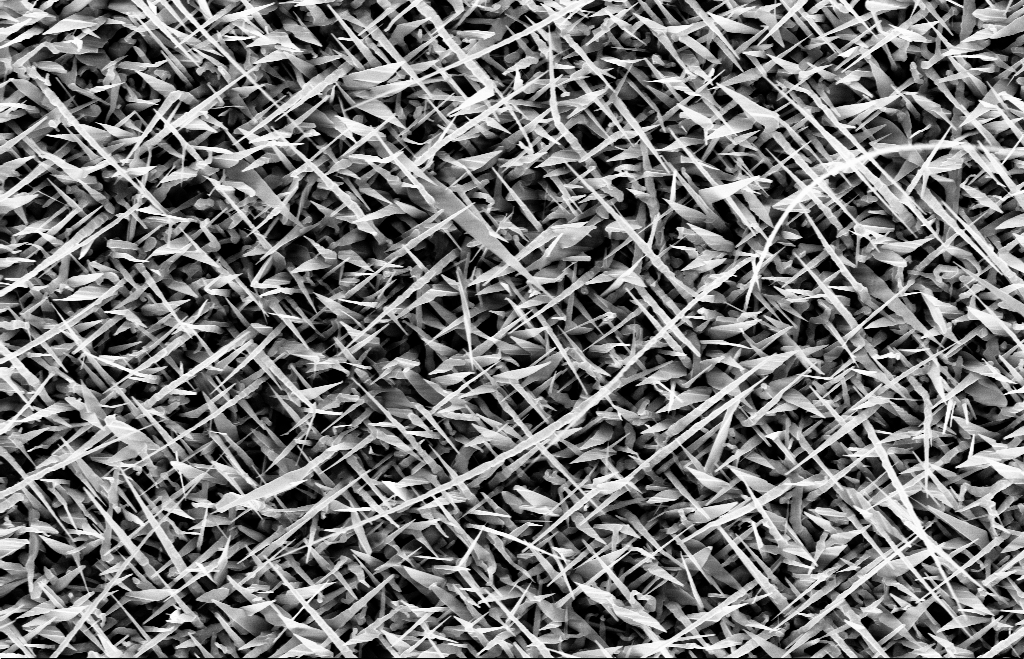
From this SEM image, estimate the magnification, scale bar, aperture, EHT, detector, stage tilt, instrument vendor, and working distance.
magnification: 20 K X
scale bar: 1000 nm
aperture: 30 µm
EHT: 10 kV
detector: InLens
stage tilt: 0°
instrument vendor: Zeiss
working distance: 8 mm